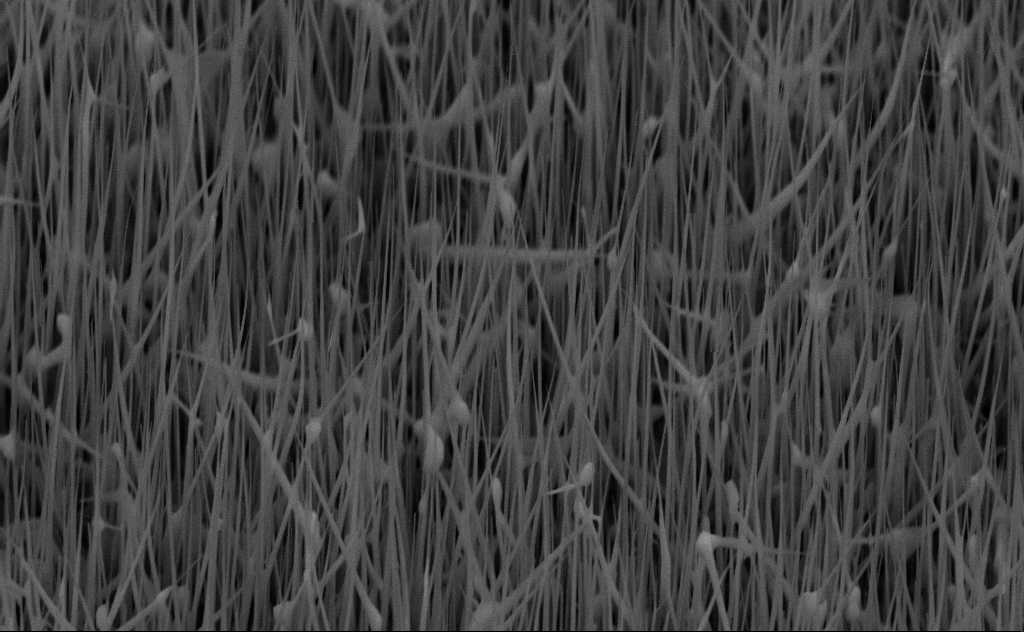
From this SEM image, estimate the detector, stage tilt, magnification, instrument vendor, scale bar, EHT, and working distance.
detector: SE2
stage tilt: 45°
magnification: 20 K X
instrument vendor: Zeiss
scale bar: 2000 nm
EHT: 10 kV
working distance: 6 mm